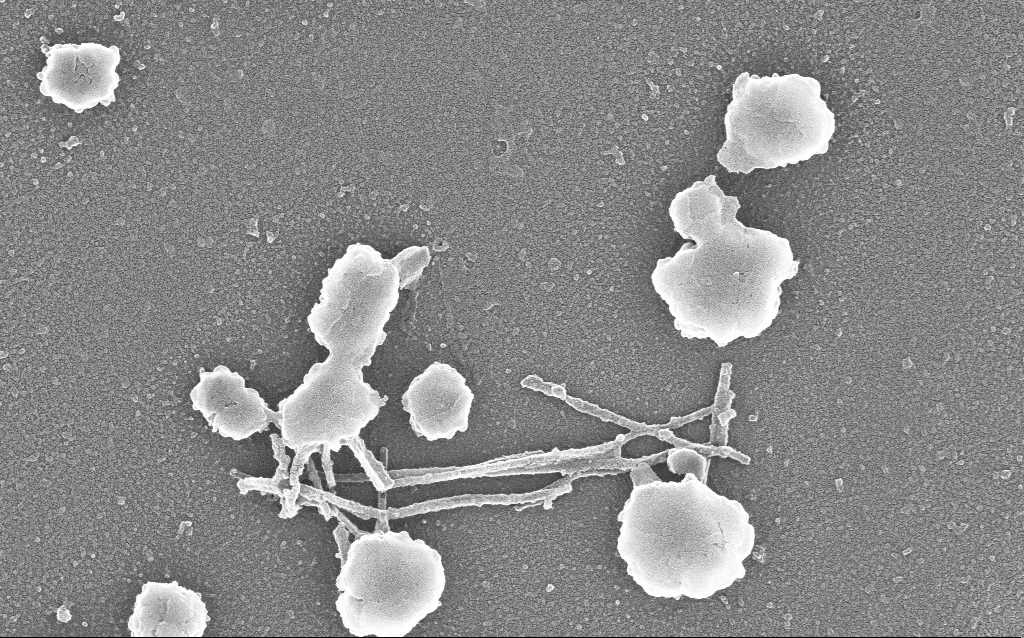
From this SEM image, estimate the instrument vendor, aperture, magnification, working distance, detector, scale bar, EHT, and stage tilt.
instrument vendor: Zeiss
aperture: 30 µm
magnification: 70 K X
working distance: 1.6 mm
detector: InLens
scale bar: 1000 nm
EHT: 20 kV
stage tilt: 0°